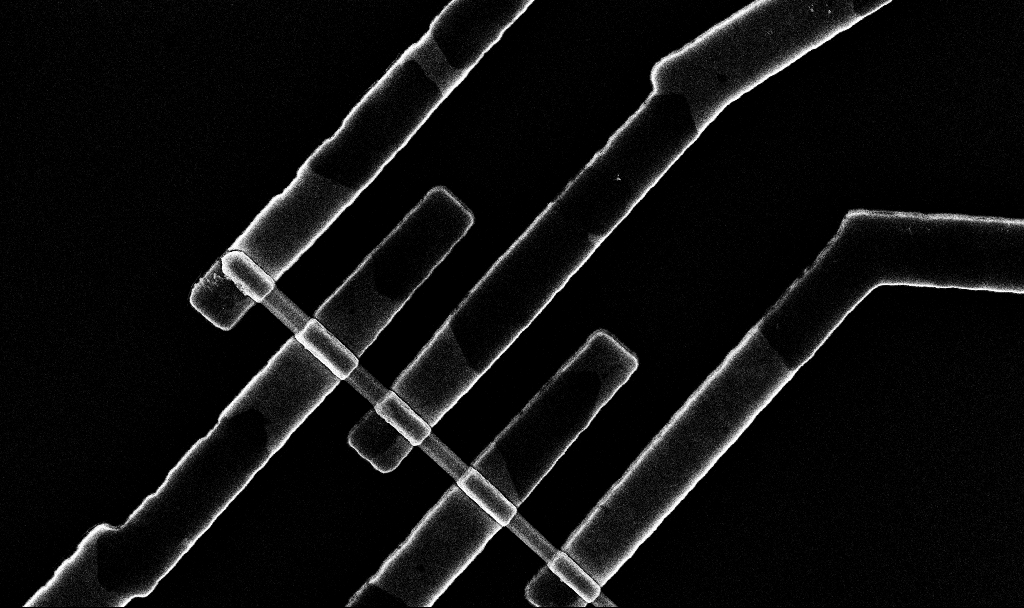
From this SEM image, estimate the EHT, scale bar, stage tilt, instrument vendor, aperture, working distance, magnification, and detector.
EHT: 10 kV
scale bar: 1000 nm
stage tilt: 0°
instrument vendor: Zeiss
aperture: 30 µm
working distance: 6.8 mm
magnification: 36.76 K X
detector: InLens